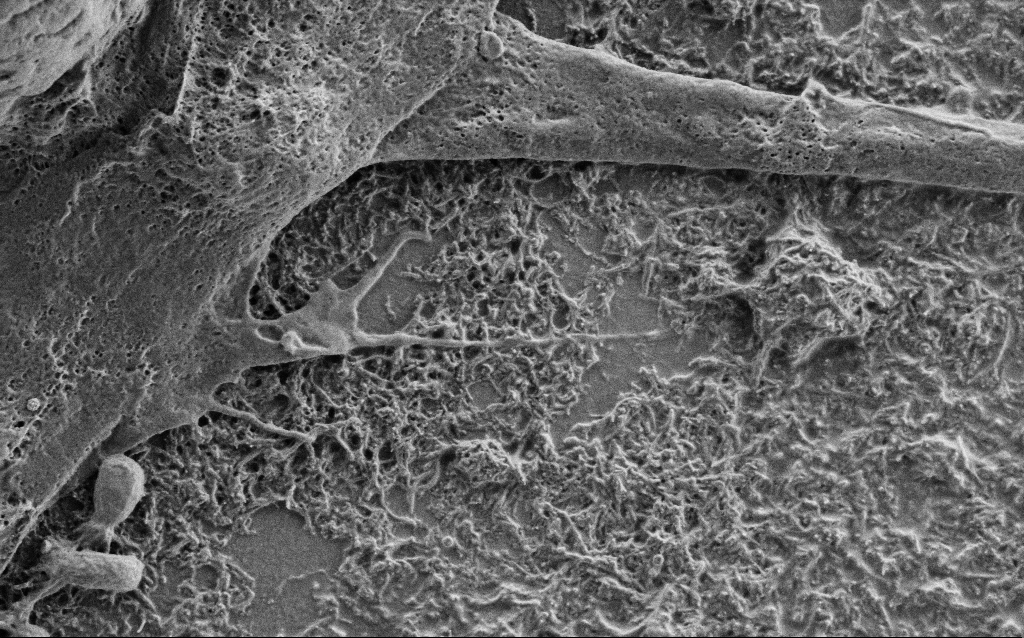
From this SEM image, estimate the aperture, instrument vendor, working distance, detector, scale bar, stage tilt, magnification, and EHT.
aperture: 30 µm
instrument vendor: Zeiss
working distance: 6 mm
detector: SE2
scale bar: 1000 nm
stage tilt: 0°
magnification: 25 K X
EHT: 1 kV